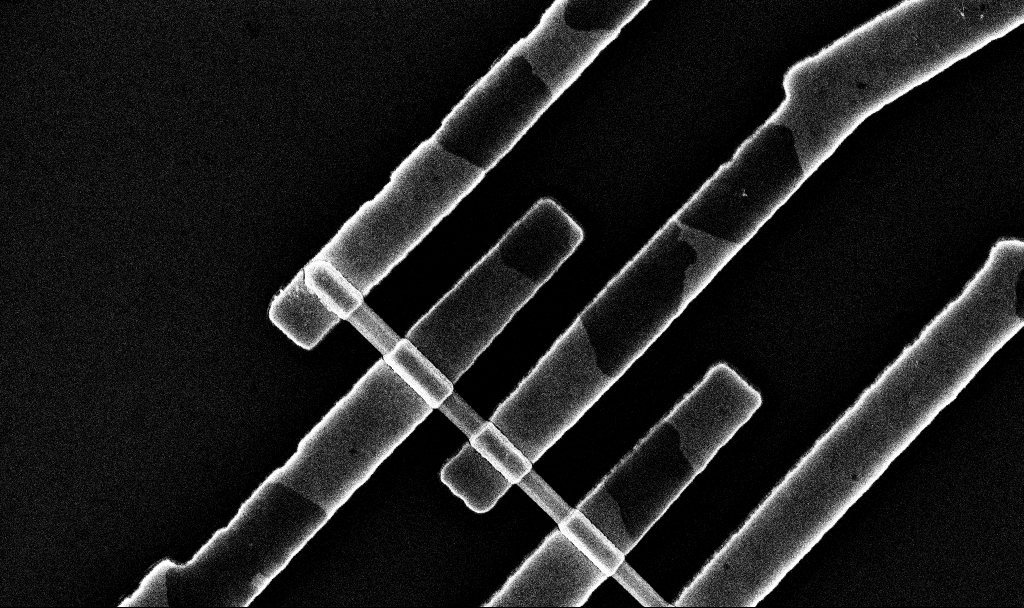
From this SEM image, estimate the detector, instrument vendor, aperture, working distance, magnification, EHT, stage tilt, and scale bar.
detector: InLens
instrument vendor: Zeiss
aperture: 30 µm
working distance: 6.7 mm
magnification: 40.64 K X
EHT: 10 kV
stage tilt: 0°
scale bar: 1000 nm